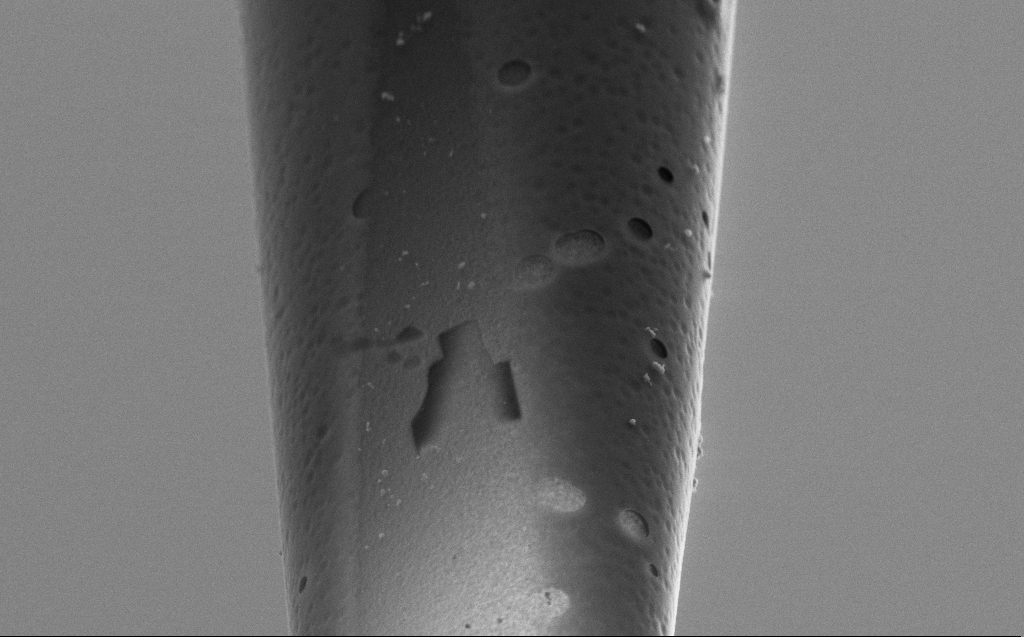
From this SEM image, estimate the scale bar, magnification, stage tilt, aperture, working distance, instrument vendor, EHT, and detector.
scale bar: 2000 nm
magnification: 16.81 K X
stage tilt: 45°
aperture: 30 µm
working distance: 4 mm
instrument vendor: Zeiss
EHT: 5 kV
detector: SE2